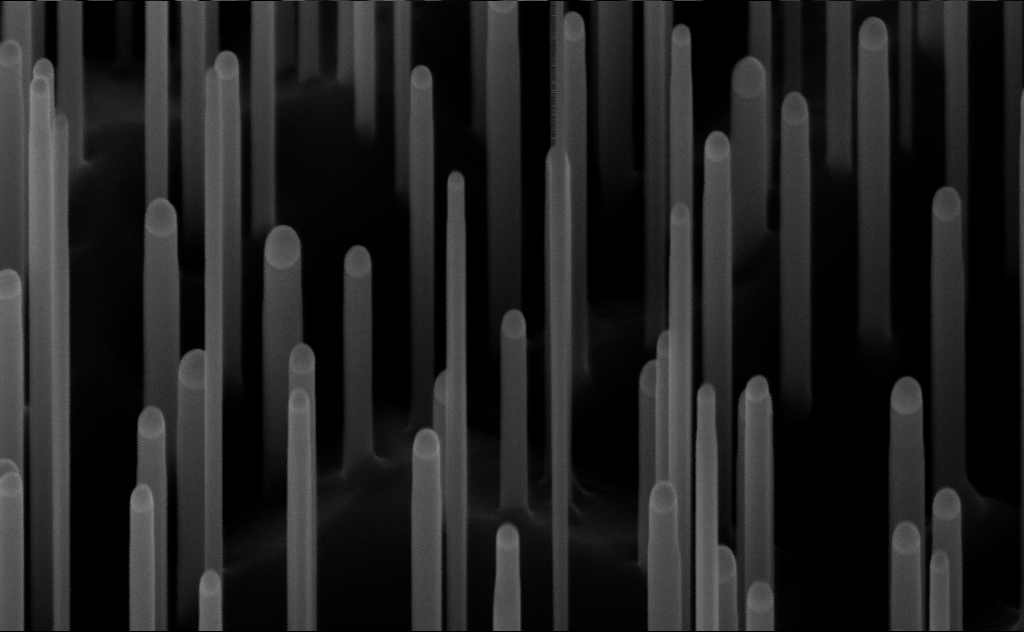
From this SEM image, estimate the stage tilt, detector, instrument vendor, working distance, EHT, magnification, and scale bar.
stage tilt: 45°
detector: InLens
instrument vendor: Zeiss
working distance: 7 mm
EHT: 10 kV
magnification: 150 K X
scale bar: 200 nm